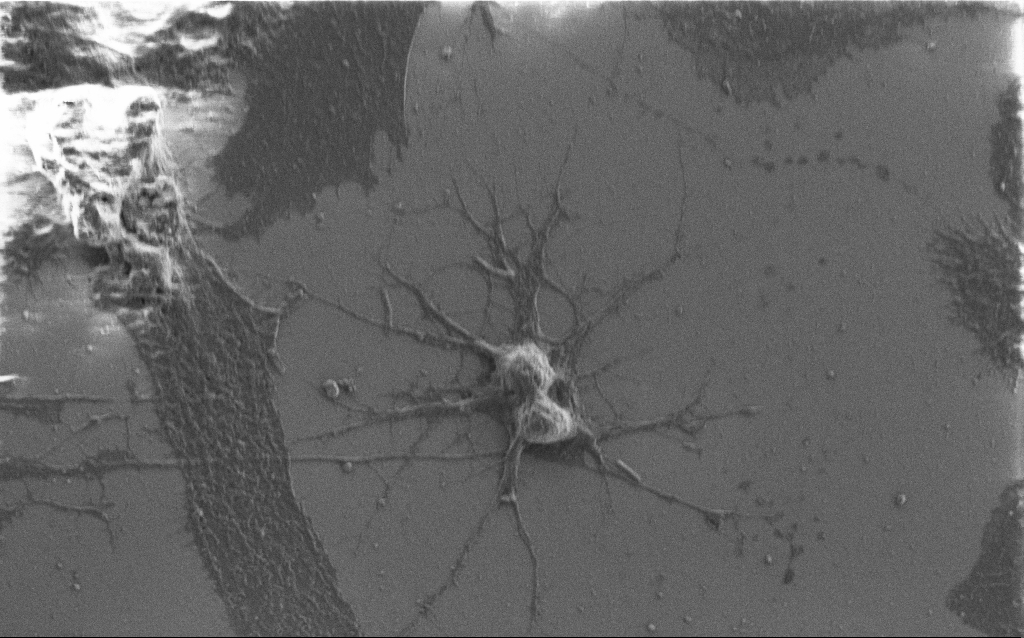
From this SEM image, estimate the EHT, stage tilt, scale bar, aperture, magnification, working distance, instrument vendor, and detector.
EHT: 1.5 kV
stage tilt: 0°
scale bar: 20000 nm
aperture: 30 µm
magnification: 3 K X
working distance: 6 mm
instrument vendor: Zeiss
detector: SE2